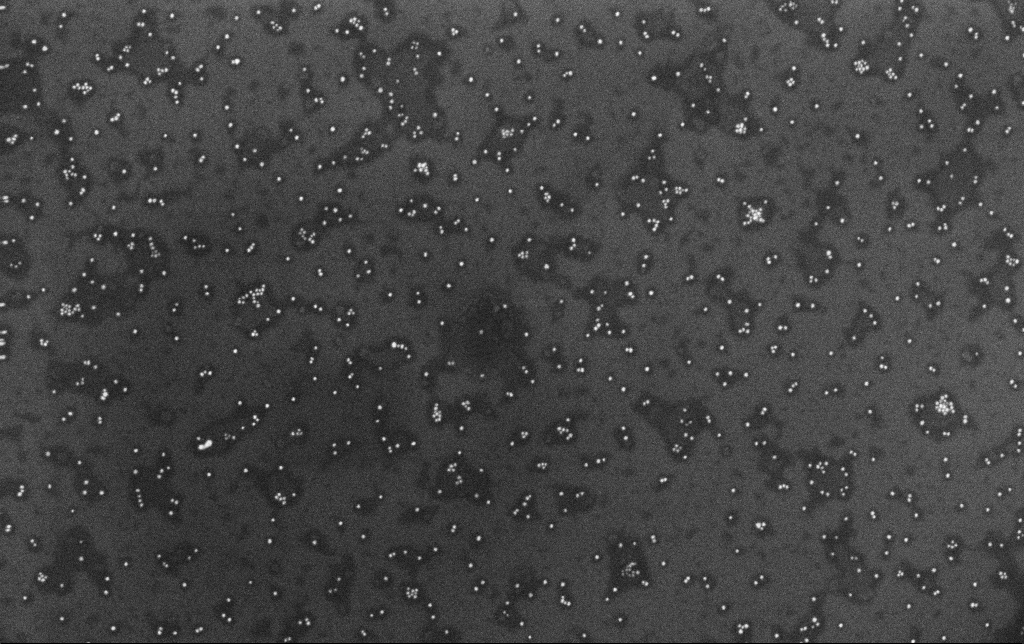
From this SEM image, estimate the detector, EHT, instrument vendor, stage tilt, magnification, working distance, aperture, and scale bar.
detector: InLens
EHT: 10 kV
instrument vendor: Zeiss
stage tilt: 0°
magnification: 100 K X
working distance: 3.4 mm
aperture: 30 µm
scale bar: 200 nm